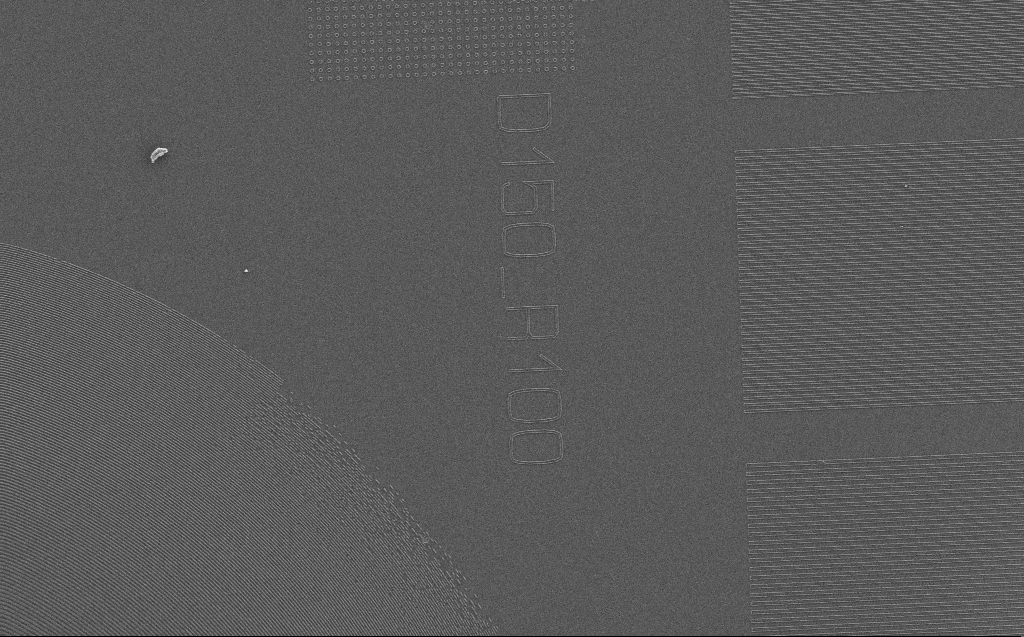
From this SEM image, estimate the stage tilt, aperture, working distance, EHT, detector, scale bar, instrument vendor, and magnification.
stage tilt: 0°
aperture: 30 µm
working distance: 4 mm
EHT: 3 kV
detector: SE2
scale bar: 10000 nm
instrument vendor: Zeiss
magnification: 4.03 K X